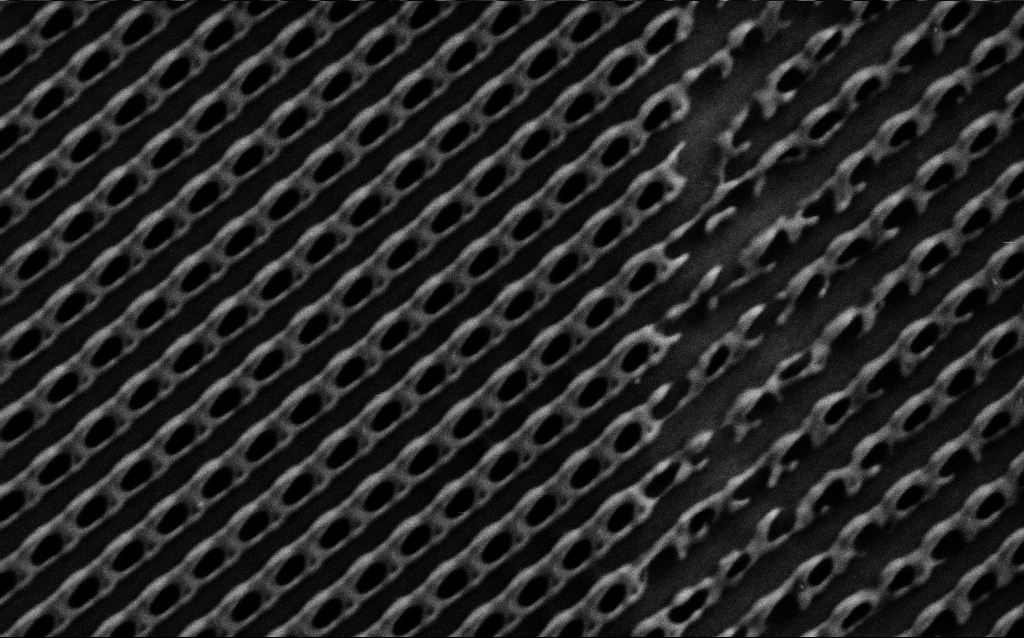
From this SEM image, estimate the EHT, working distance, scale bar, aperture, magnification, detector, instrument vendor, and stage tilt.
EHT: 1.5 kV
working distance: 8 mm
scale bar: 1000 nm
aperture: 30 µm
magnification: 45.26 K X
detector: SE2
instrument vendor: Zeiss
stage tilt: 0°